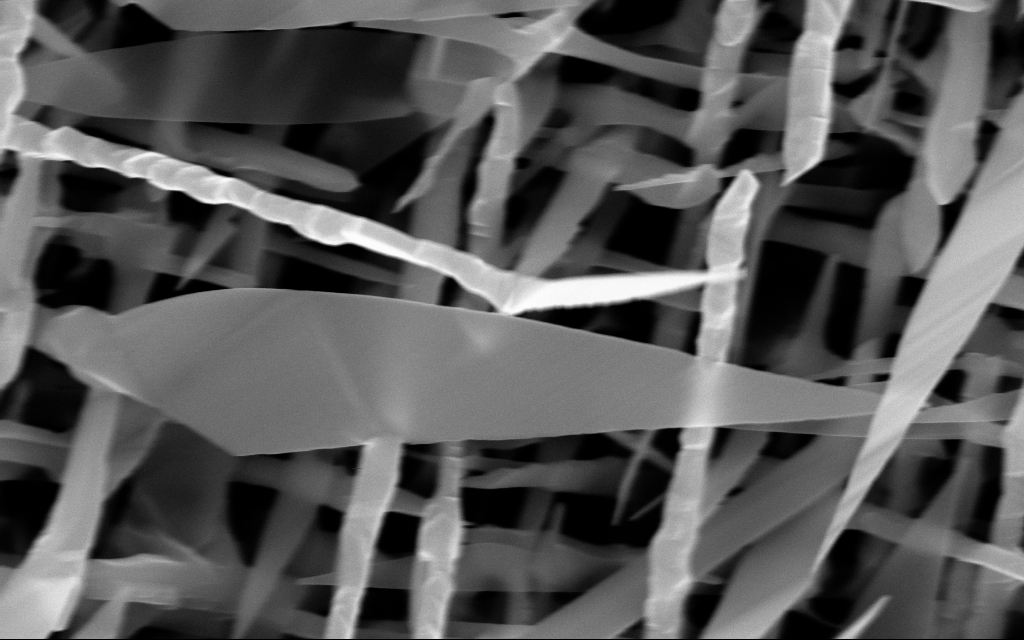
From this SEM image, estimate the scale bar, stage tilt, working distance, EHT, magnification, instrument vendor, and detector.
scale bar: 200 nm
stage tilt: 0°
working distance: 7 mm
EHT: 10 kV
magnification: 76.74 K X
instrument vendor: Zeiss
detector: InLens